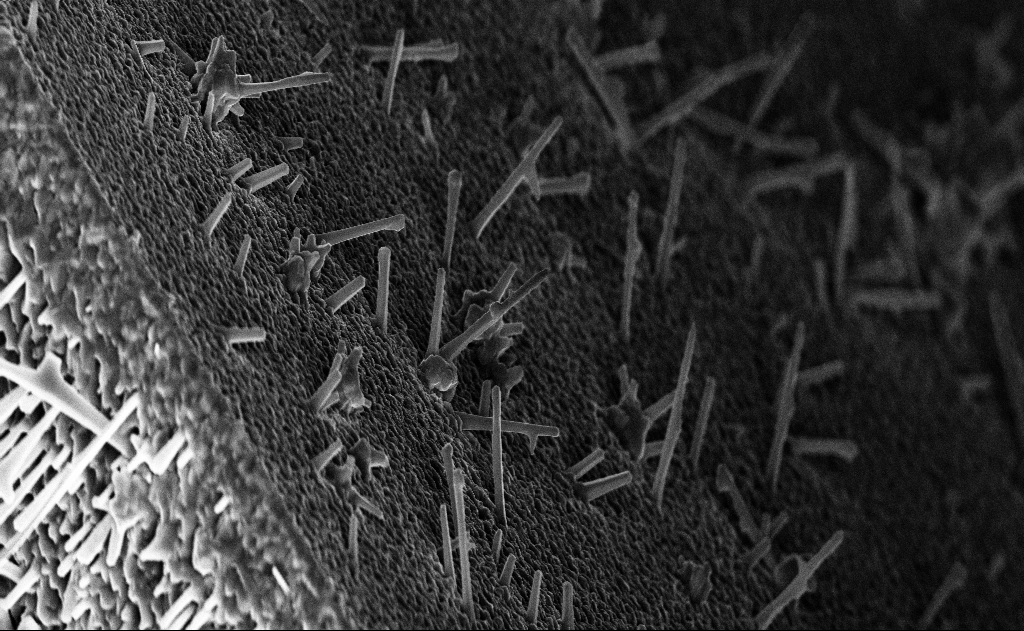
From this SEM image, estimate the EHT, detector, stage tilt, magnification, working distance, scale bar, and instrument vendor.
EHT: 10 kV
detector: InLens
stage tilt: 0°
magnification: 10 K X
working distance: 15 mm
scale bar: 2000 nm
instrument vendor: Zeiss